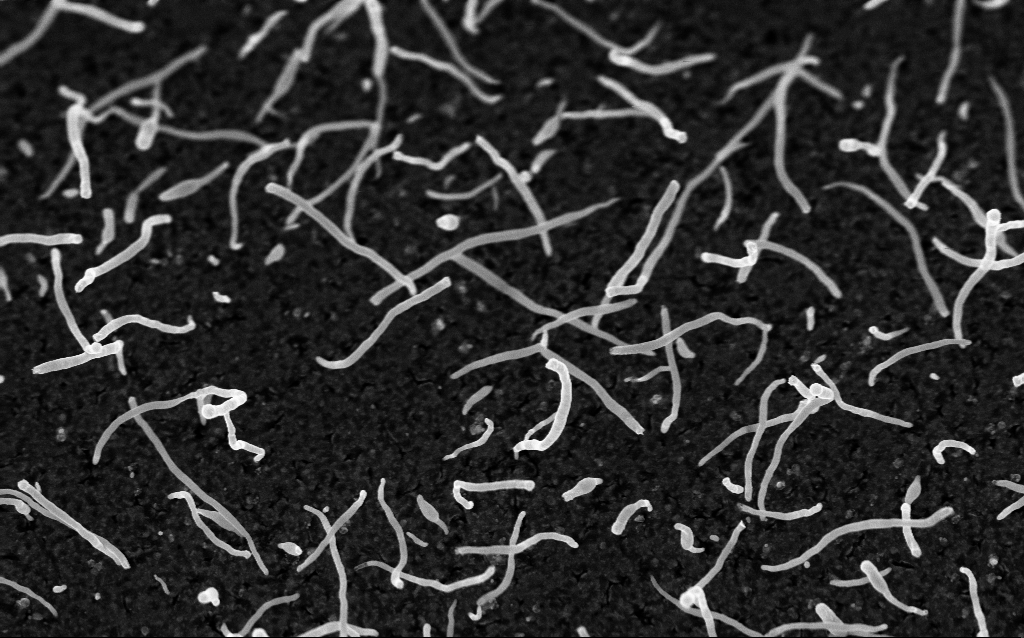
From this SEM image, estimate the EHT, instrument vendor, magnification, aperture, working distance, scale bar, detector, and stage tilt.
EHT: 5 kV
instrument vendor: Zeiss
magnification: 50 K X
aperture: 30 µm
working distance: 1.7 mm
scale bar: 1000 nm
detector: InLens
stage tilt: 0°